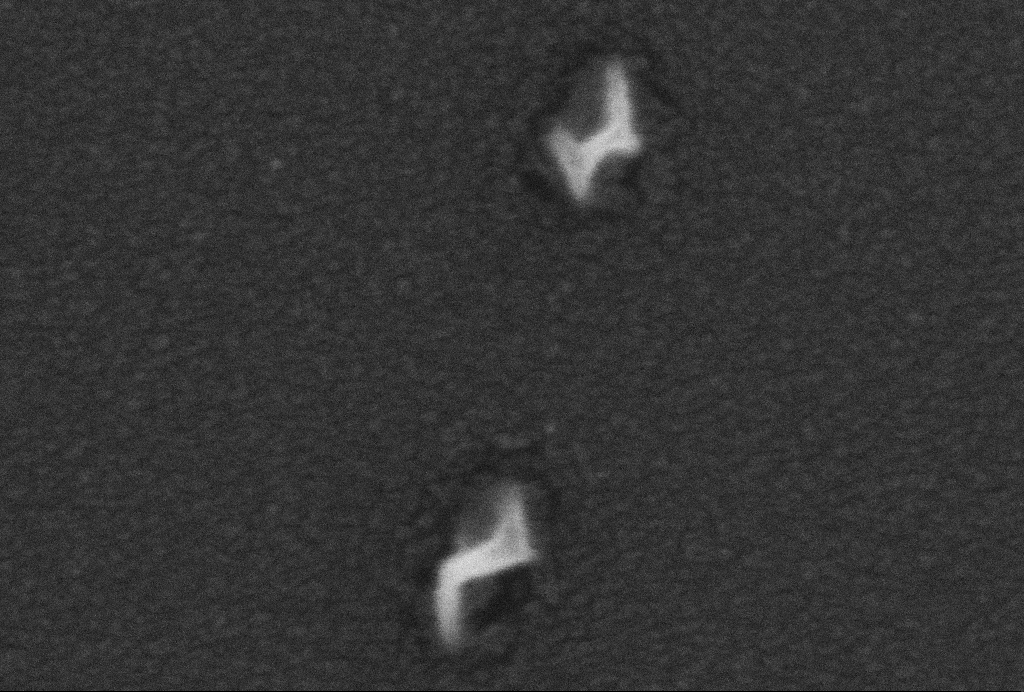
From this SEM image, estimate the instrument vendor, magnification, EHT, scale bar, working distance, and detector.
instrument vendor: Zeiss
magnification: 500 K X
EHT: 5 kV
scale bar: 100 nm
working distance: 2.6 mm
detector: SE2